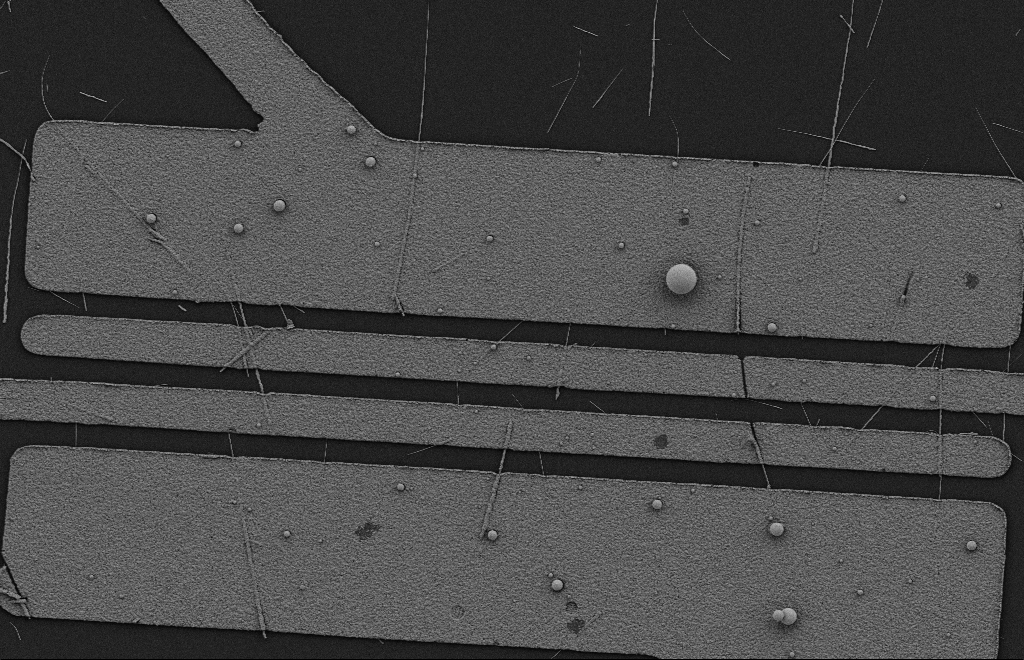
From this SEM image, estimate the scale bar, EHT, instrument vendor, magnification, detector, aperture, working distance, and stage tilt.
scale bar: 2000 nm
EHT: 2 kV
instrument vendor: Zeiss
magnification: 5.97 K X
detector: SE2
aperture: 20 µm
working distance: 10 mm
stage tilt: -0.3°